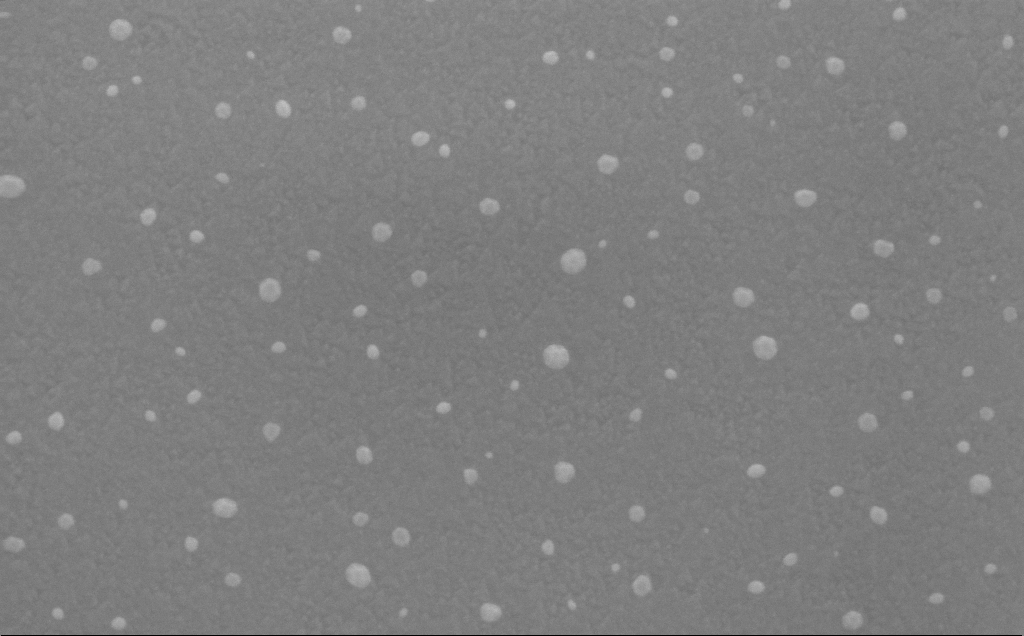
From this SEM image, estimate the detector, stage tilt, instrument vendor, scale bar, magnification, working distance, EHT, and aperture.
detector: InLens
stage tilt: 15.5°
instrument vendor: Zeiss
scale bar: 200 nm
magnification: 143.2 K X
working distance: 5 mm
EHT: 10 kV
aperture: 30 µm